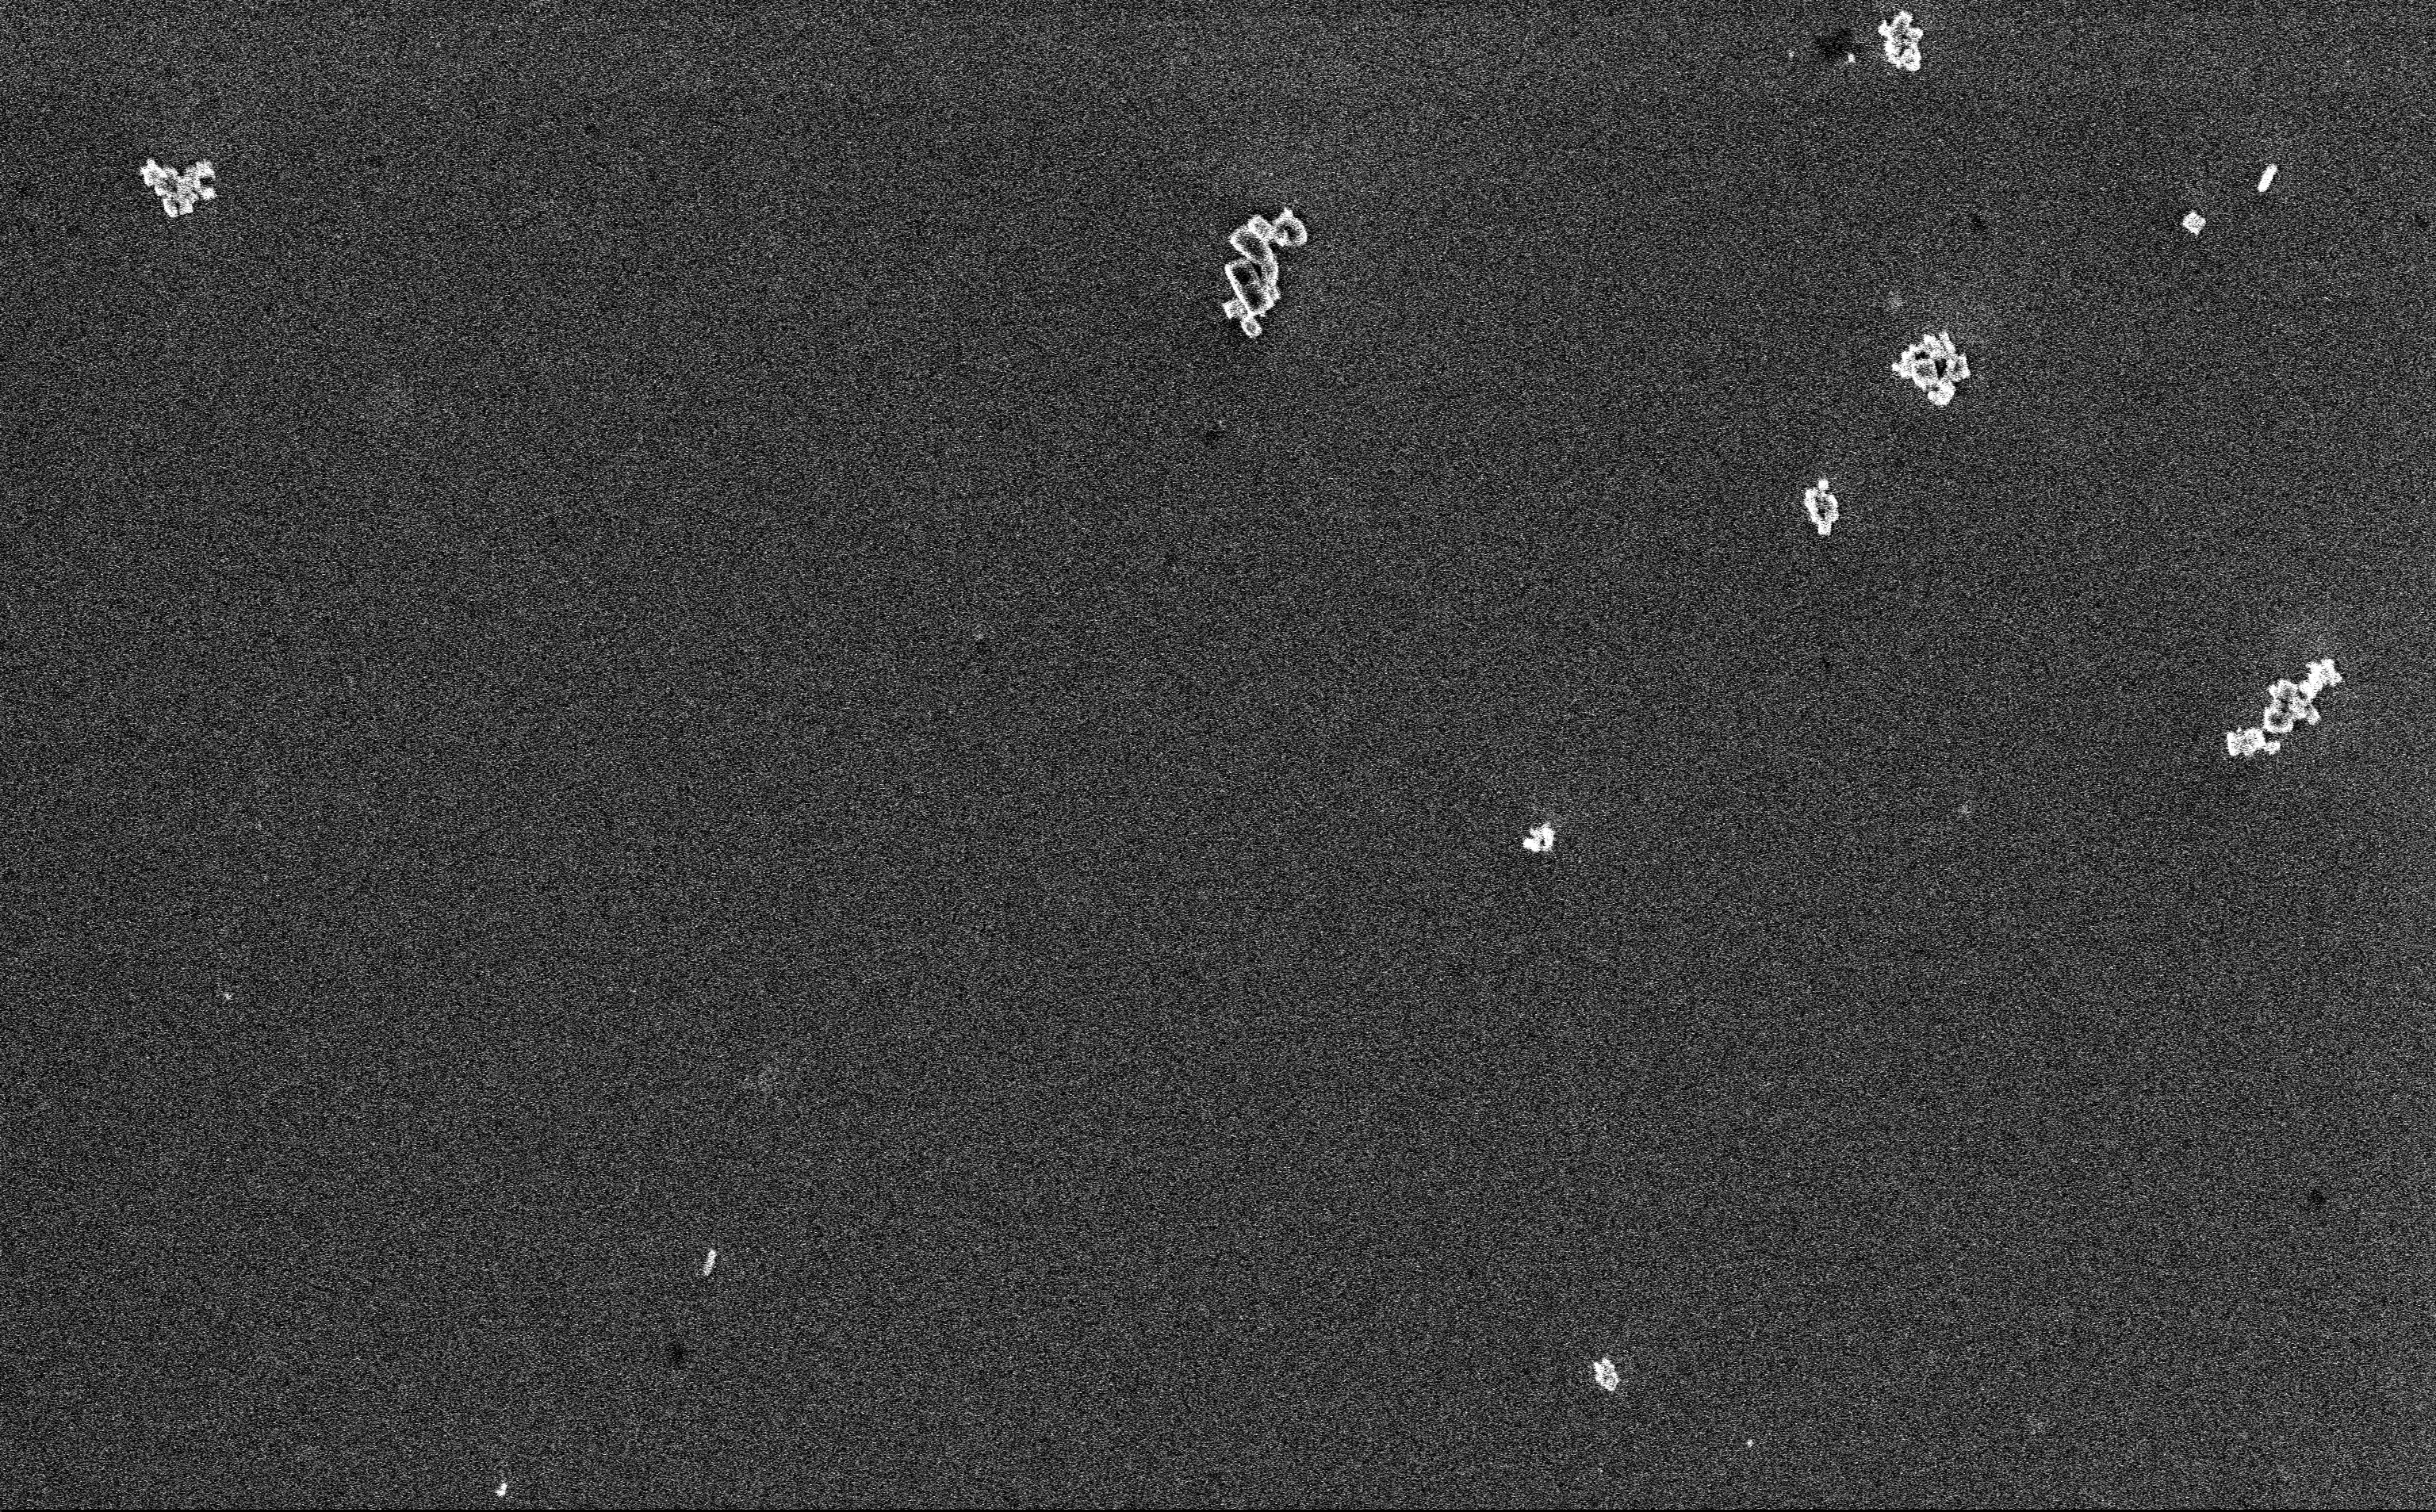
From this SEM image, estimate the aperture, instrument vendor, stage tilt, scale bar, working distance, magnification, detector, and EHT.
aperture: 30 µm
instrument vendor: Zeiss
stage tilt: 0°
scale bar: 2000 nm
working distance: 3 mm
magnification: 12.85 K X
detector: InLens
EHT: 3 kV